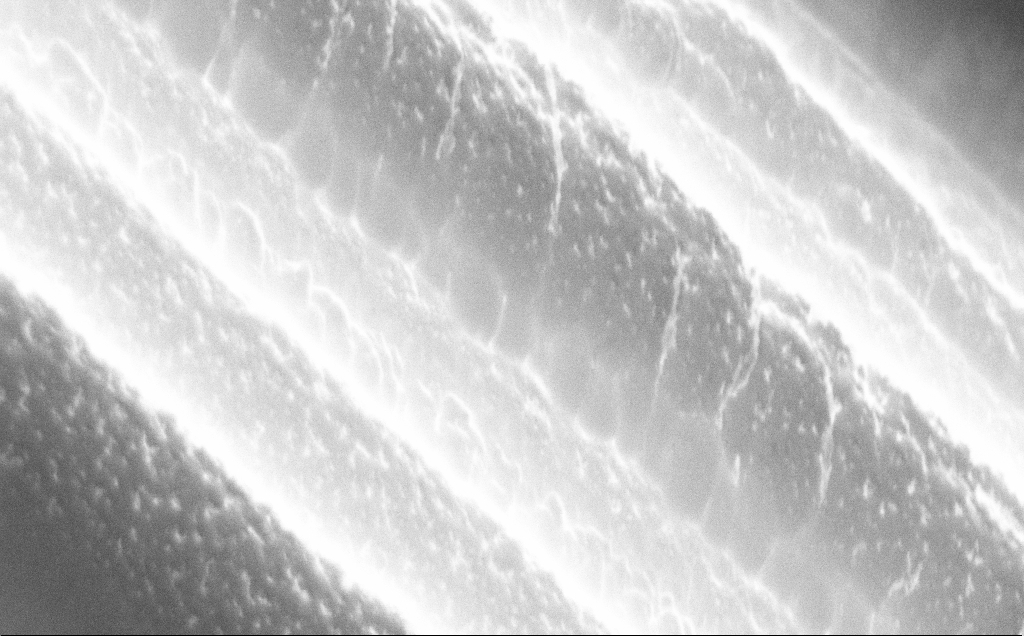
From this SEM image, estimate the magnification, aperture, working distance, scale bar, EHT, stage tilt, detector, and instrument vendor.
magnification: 140.18 K X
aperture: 30 µm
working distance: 9 mm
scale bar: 200 nm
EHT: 10 kV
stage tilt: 50°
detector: InLens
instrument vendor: Zeiss